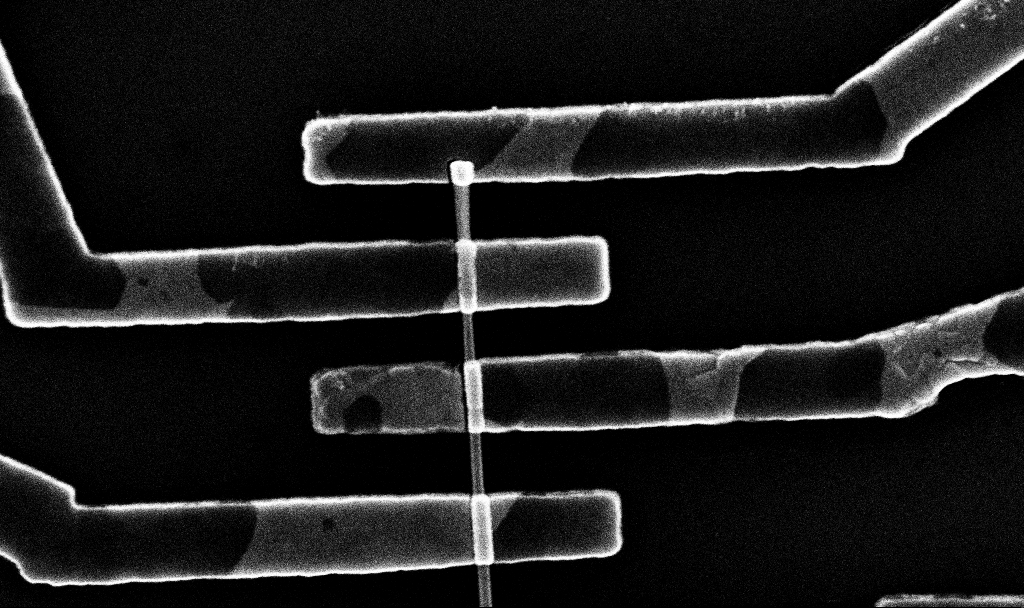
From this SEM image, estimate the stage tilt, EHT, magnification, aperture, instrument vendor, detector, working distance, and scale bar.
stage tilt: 0°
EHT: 10 kV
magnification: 43.41 K X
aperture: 30 µm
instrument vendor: Zeiss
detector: InLens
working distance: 6.8 mm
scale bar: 1000 nm